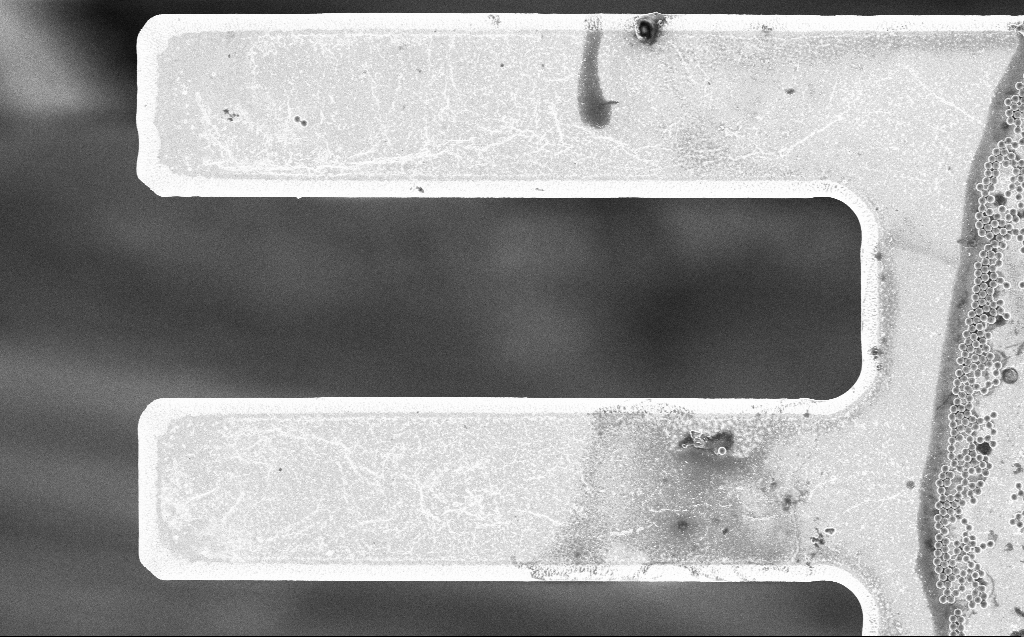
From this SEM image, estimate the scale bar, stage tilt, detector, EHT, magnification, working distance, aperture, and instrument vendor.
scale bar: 20000 nm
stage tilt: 0°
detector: InLens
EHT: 3 kV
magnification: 3.54 K X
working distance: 7 mm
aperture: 30 µm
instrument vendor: Zeiss